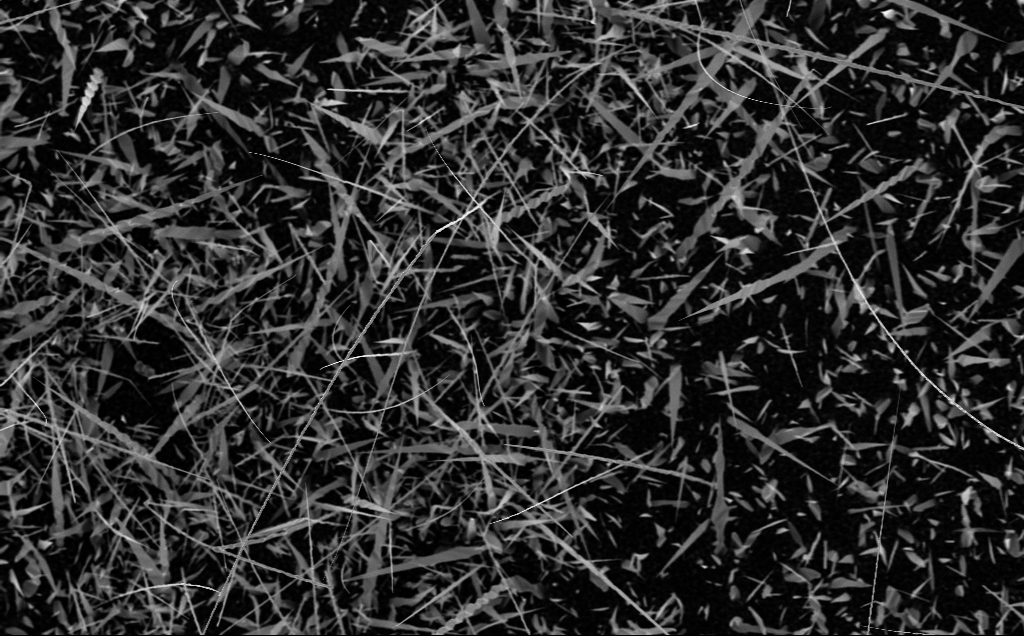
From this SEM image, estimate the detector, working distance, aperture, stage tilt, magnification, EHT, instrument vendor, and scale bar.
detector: InLens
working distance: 6 mm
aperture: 30 µm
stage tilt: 0°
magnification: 5 K X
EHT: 10 kV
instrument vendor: Zeiss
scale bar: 10000 nm